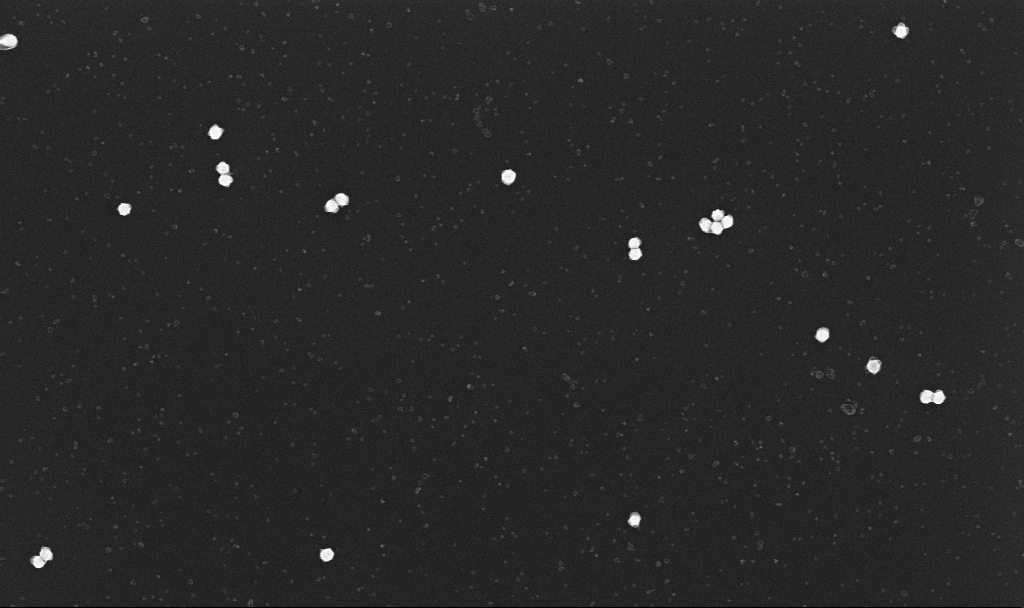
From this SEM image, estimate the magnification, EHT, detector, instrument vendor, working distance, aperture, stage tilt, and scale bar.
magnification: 70 K X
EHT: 10 kV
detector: InLens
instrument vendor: Zeiss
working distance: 3.4 mm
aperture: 30 µm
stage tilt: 0°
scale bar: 1000 nm